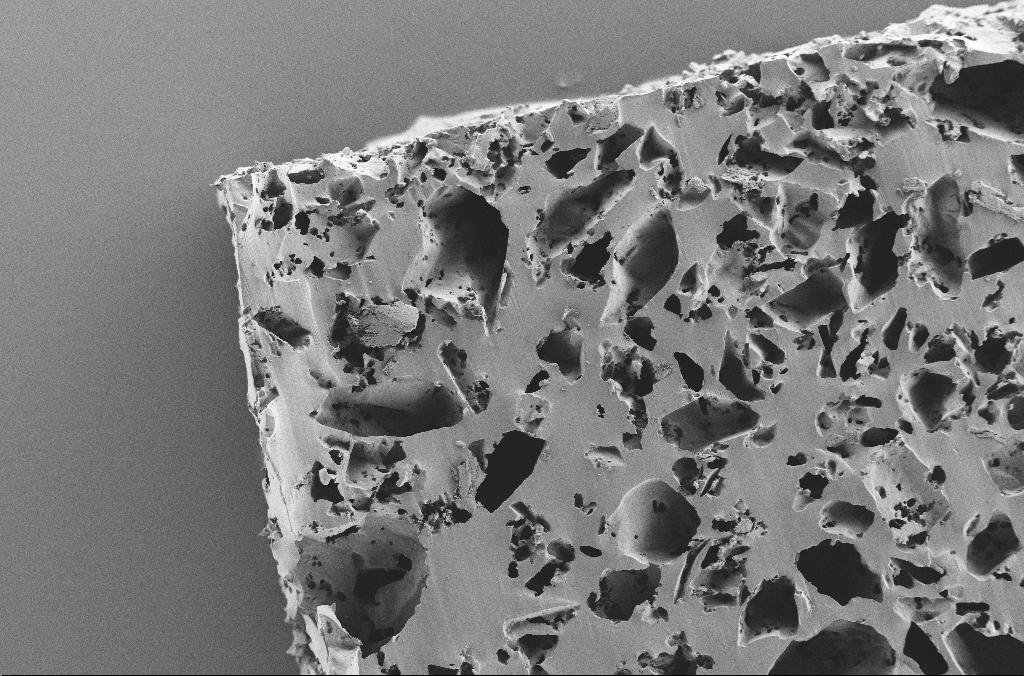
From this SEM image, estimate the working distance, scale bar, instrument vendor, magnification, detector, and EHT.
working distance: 3.1 mm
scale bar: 100000 nm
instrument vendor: Zeiss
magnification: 0.25 K X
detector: SE2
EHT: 2 kV